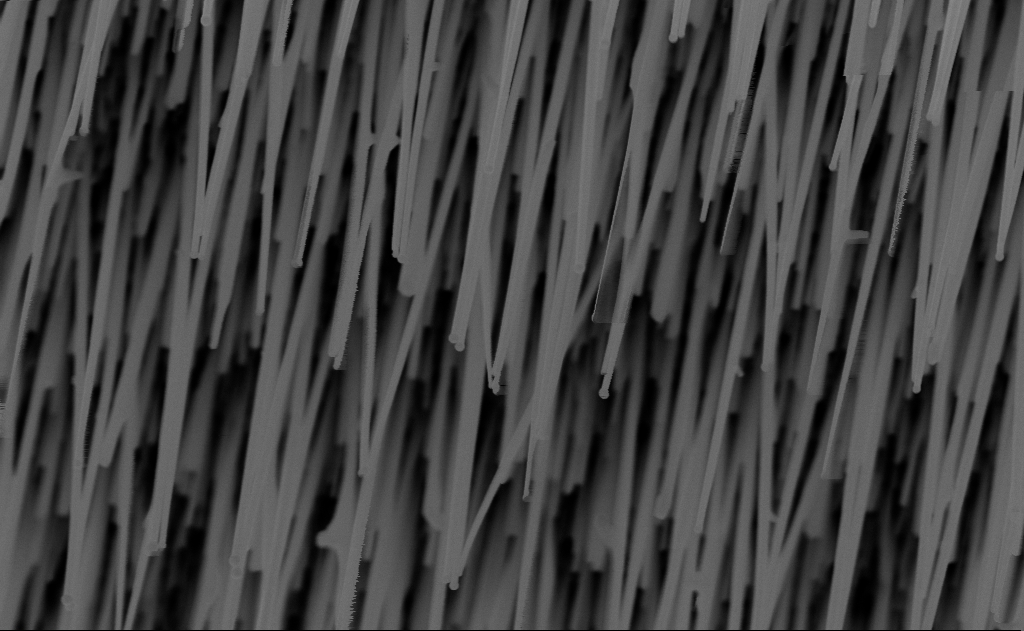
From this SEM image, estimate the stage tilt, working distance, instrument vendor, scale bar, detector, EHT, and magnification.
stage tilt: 0°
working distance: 9 mm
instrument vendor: Zeiss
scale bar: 1000 nm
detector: InLens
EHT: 10 kV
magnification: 40 K X